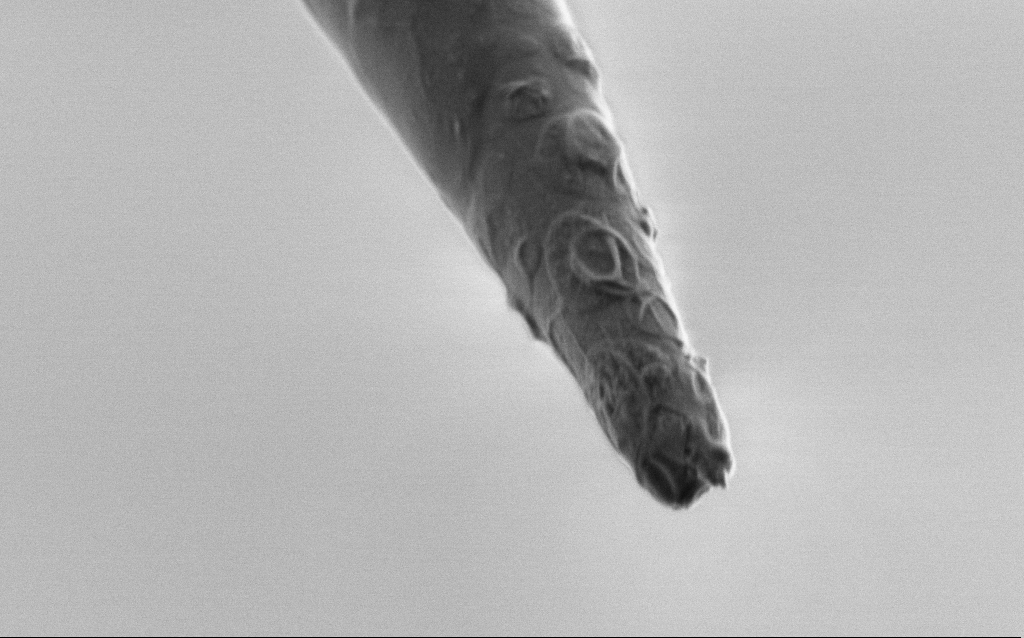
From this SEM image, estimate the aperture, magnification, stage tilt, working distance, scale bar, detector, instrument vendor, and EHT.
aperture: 30 µm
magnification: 50 K X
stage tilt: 45°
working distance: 6 mm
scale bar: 1000 nm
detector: SE2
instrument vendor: Zeiss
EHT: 1 kV